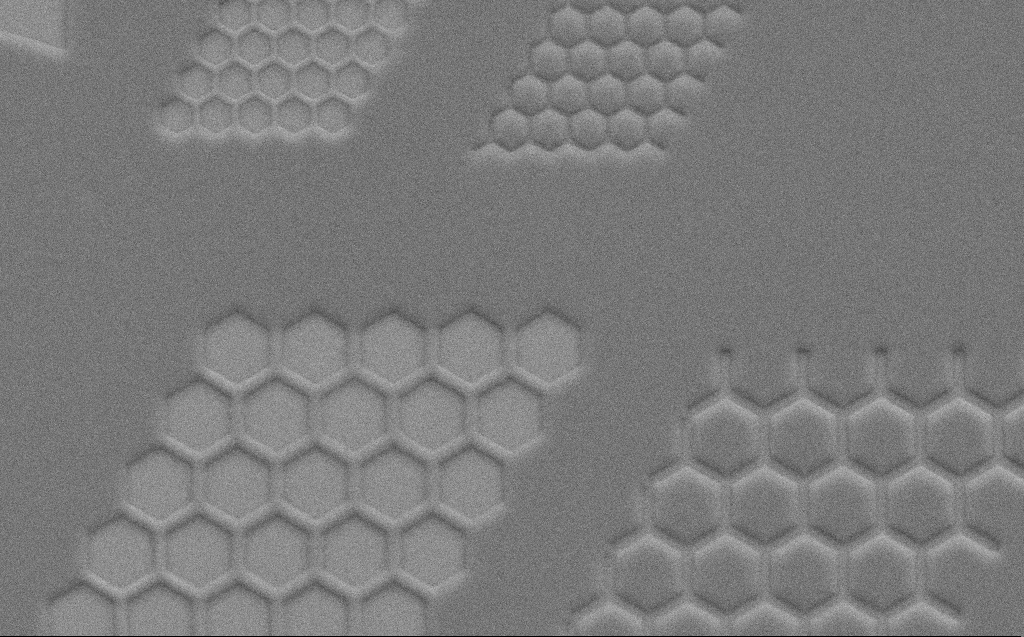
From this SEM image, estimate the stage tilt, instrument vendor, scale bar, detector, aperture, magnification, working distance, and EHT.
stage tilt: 45°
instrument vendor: Zeiss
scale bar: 10000 nm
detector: SE2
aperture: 30 µm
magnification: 4.63 K X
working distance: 6 mm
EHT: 10 kV